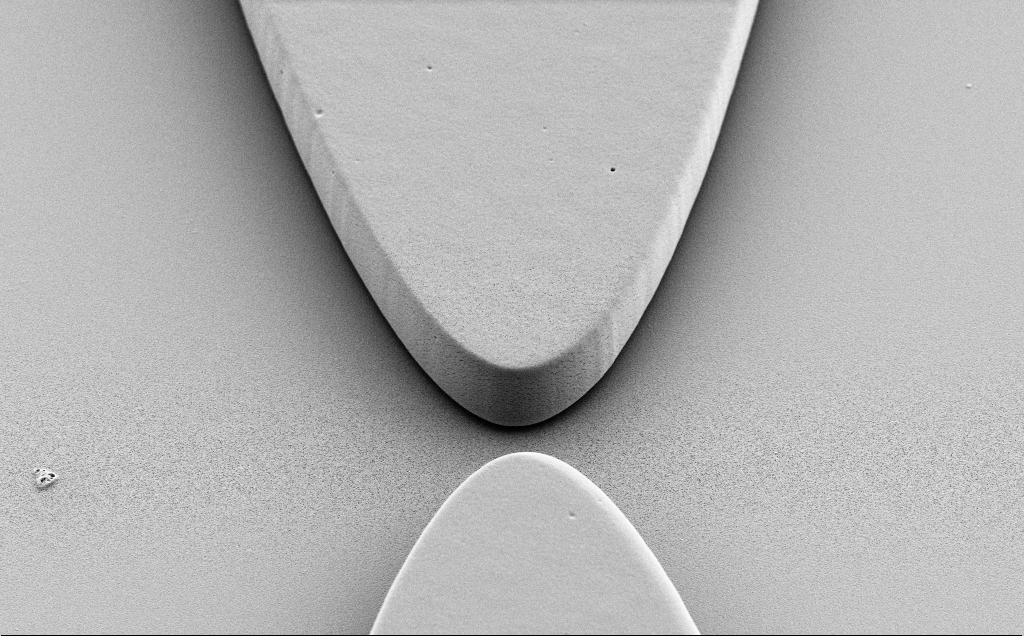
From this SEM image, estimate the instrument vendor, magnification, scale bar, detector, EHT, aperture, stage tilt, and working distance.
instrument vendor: Zeiss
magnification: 6.15 K X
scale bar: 10000 nm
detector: SE2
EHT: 5 kV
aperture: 30 µm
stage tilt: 40°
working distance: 9 mm